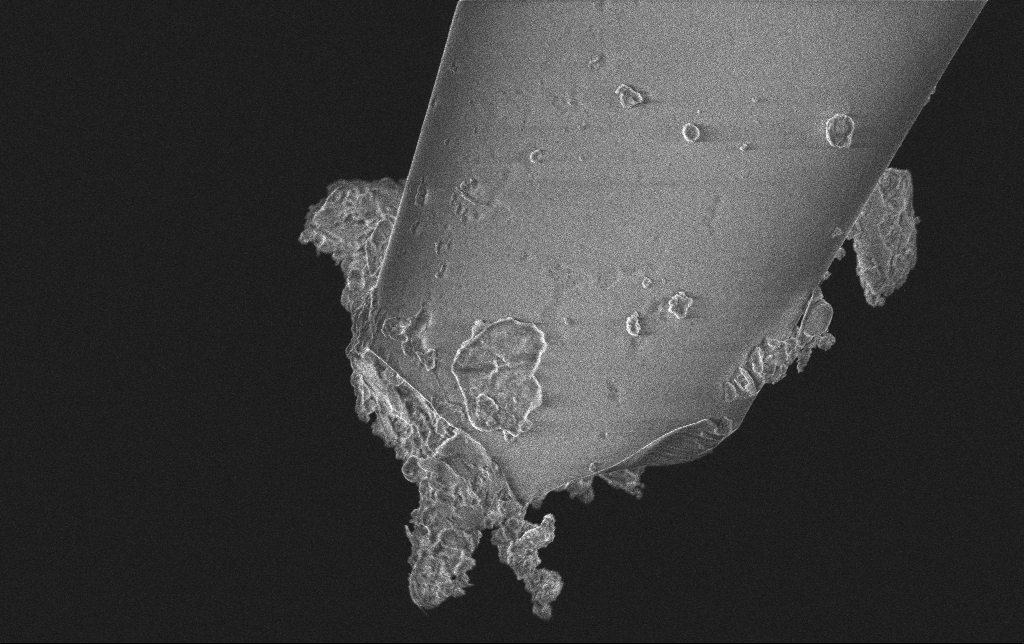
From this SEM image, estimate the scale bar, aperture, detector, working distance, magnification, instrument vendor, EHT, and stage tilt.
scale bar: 2000 nm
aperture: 30 µm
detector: InLens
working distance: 6.2 mm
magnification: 16.96 K X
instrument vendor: Zeiss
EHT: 2 kV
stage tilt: -0.3°